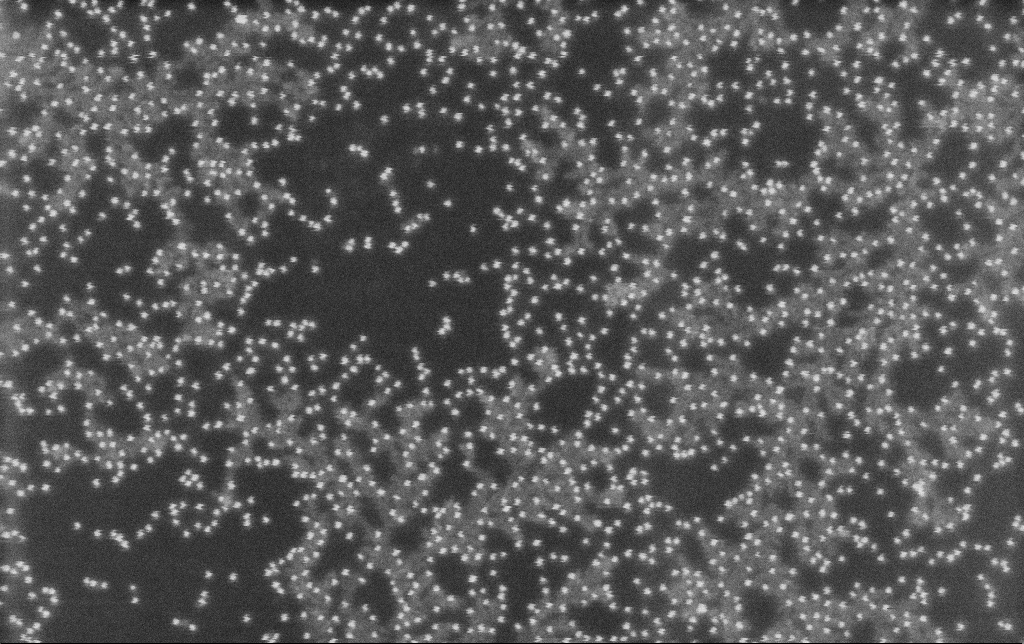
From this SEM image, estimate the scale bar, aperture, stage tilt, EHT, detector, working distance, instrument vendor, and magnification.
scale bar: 100 nm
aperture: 30 µm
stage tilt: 0°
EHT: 10 kV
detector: SE2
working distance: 11.3 mm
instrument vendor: Zeiss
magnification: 175.72 K X